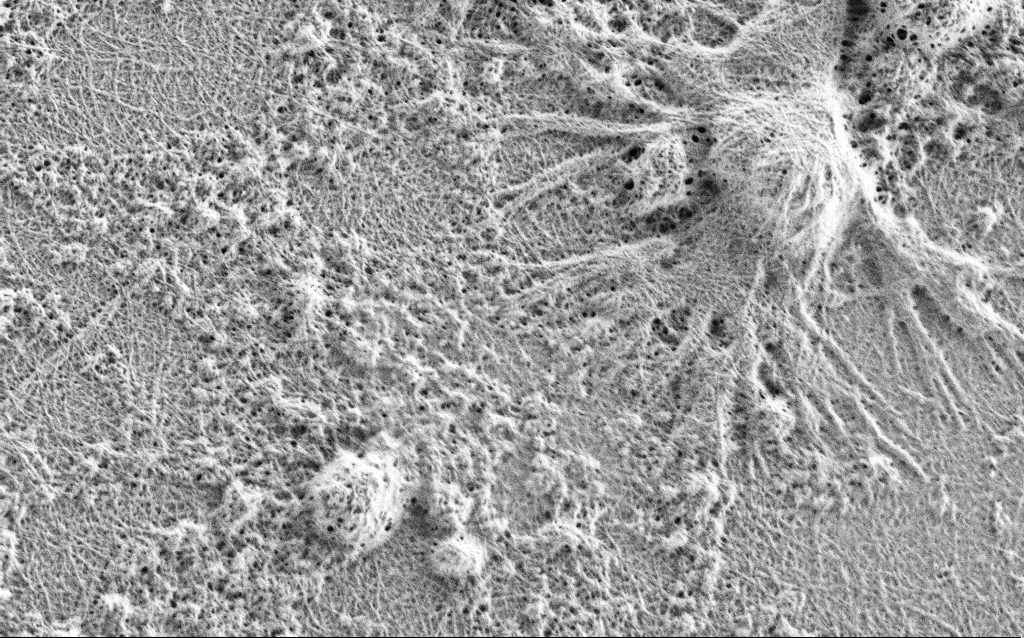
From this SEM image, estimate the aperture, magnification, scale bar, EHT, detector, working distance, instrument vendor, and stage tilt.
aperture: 30 µm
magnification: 15 K X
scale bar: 2000 nm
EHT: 0.9 kV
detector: SE2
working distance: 4 mm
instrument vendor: Zeiss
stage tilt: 0°